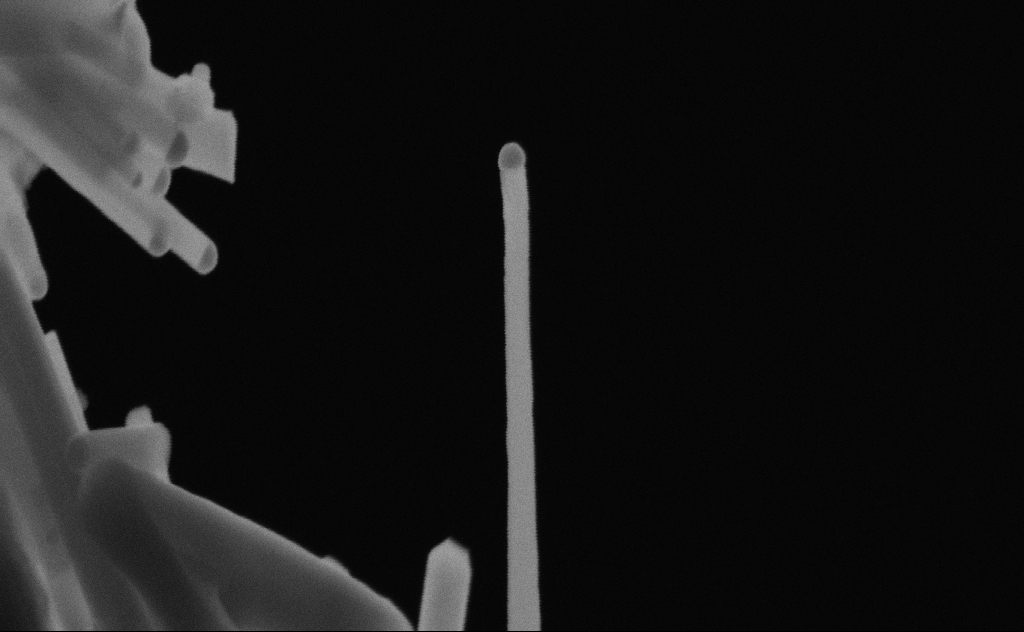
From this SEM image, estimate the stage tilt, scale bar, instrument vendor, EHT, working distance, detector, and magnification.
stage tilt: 0°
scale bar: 200 nm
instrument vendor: Zeiss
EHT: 20 kV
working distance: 9 mm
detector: SE2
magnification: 209.47 K X